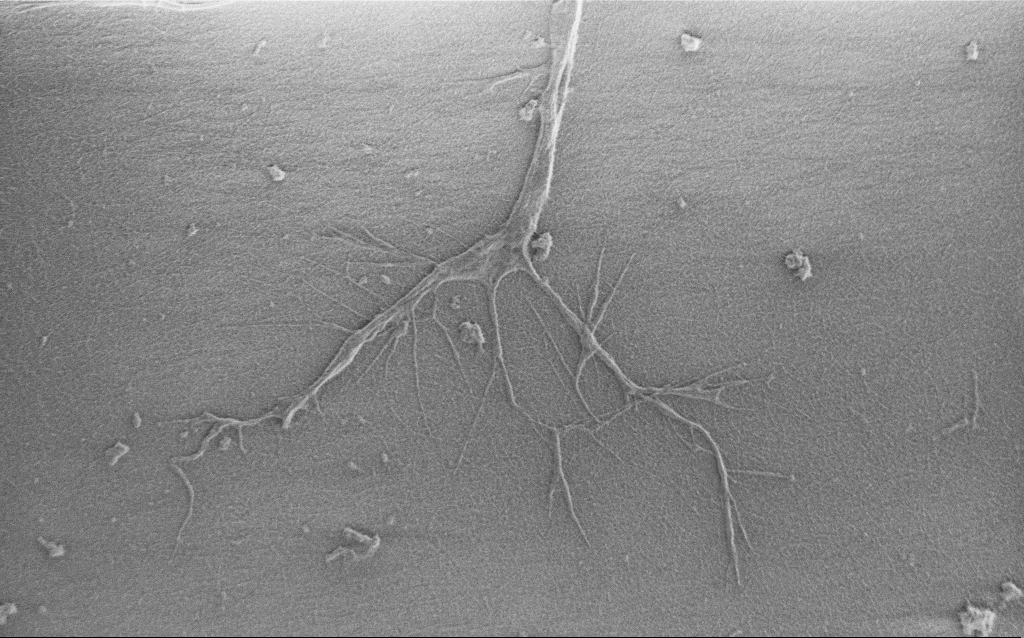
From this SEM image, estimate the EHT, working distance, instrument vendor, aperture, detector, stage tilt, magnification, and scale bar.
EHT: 0.9 kV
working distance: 7 mm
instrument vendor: Zeiss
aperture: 30 µm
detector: SE2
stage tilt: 0°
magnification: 6 K X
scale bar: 10000 nm